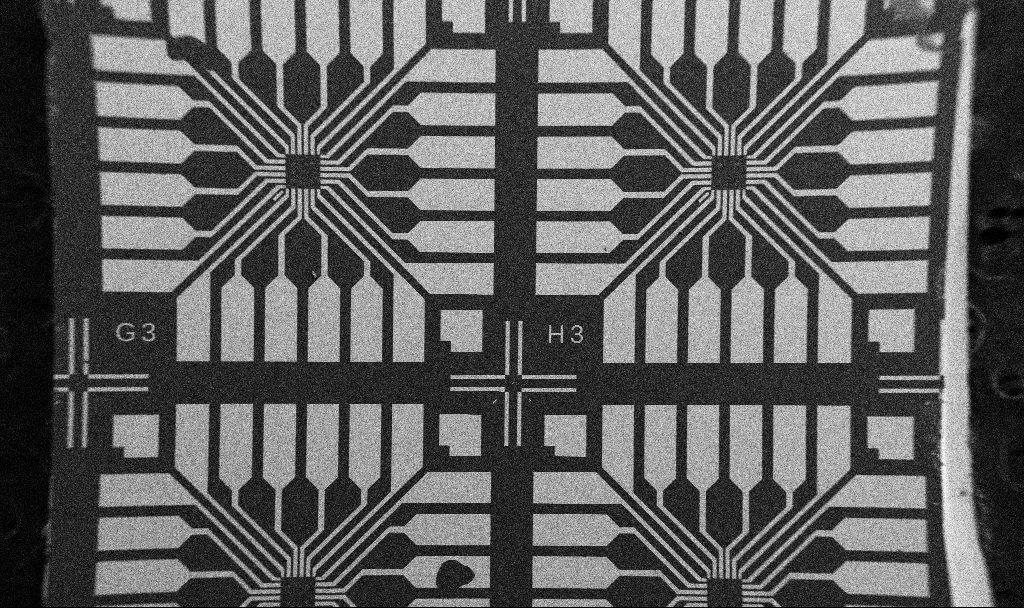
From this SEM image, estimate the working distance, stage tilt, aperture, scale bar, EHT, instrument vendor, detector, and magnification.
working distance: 10.7 mm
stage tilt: -0°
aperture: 30 µm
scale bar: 200000 nm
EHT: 5 kV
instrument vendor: Zeiss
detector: SE2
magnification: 0.077 K X